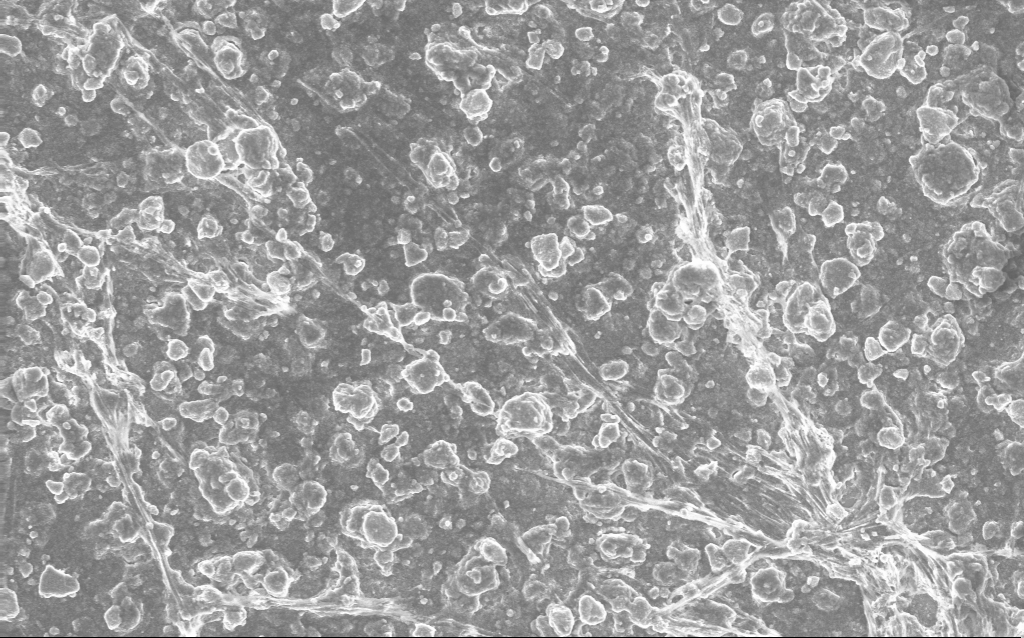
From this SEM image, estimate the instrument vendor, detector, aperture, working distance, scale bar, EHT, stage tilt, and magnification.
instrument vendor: Zeiss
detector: InLens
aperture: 30 µm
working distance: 2.7 mm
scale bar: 10000 nm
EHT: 10 kV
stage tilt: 0°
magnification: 1.69 K X